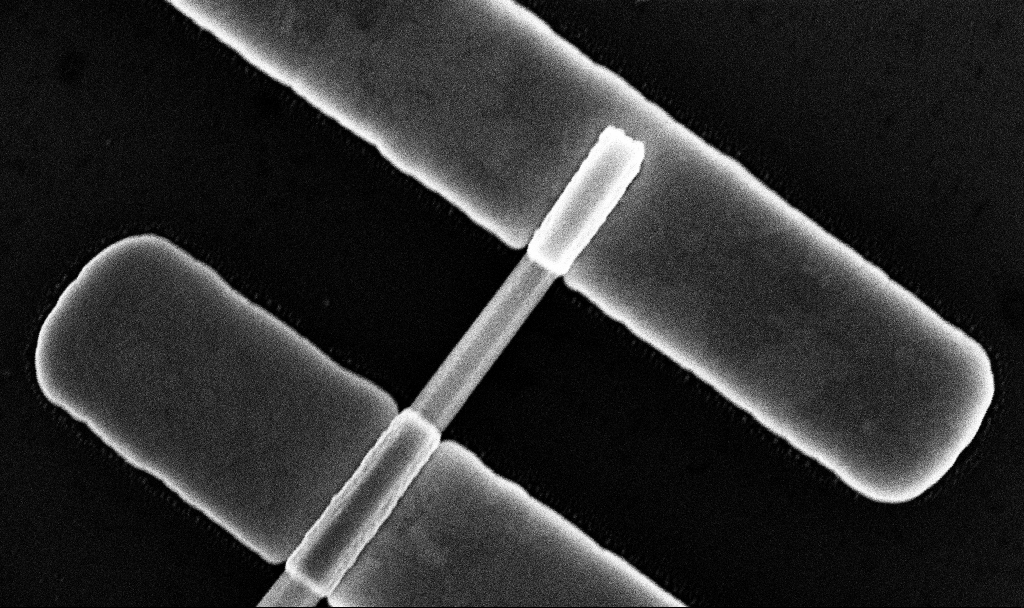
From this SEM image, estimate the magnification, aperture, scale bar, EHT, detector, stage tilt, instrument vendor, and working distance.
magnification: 104.55 K X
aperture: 30 µm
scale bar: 200 nm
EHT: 10 kV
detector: InLens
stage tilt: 0°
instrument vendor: Zeiss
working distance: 7.8 mm